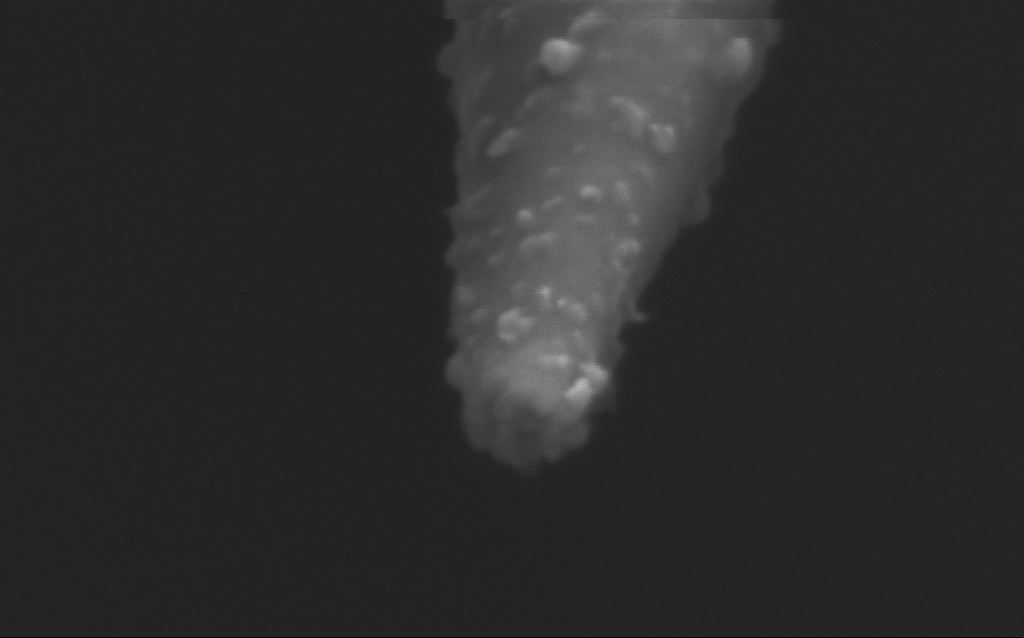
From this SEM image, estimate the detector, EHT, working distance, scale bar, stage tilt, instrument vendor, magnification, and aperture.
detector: InLens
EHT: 2.5 kV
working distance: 5 mm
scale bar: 100 nm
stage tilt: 45°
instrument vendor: Zeiss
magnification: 500 K X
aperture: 30 µm